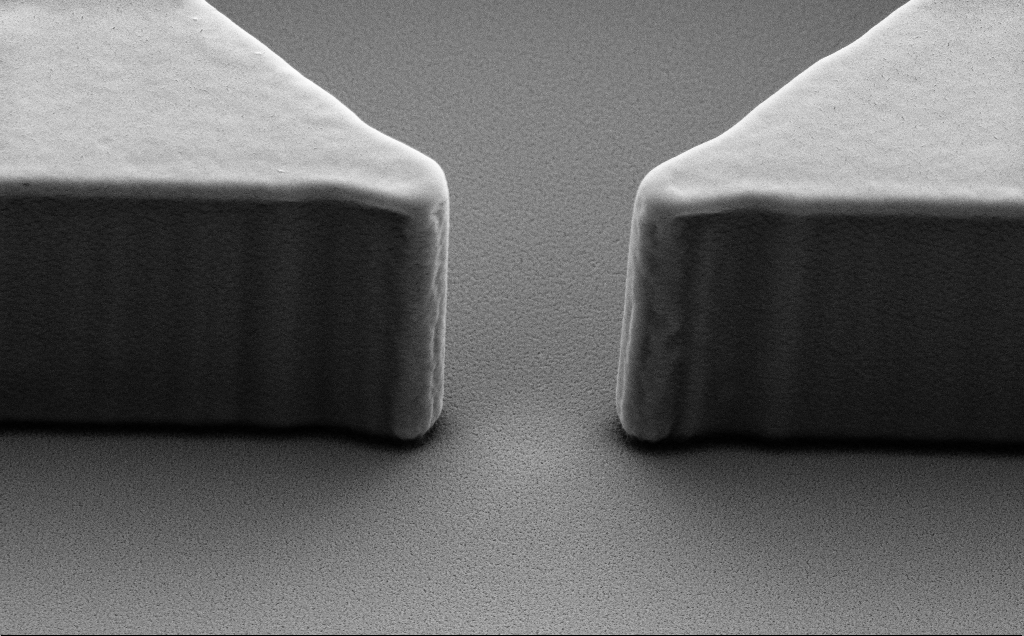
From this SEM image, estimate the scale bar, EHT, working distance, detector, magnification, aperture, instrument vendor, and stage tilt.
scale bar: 2000 nm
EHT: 5 kV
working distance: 8 mm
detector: SE2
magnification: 17.76 K X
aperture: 30 µm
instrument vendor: Zeiss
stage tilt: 40°